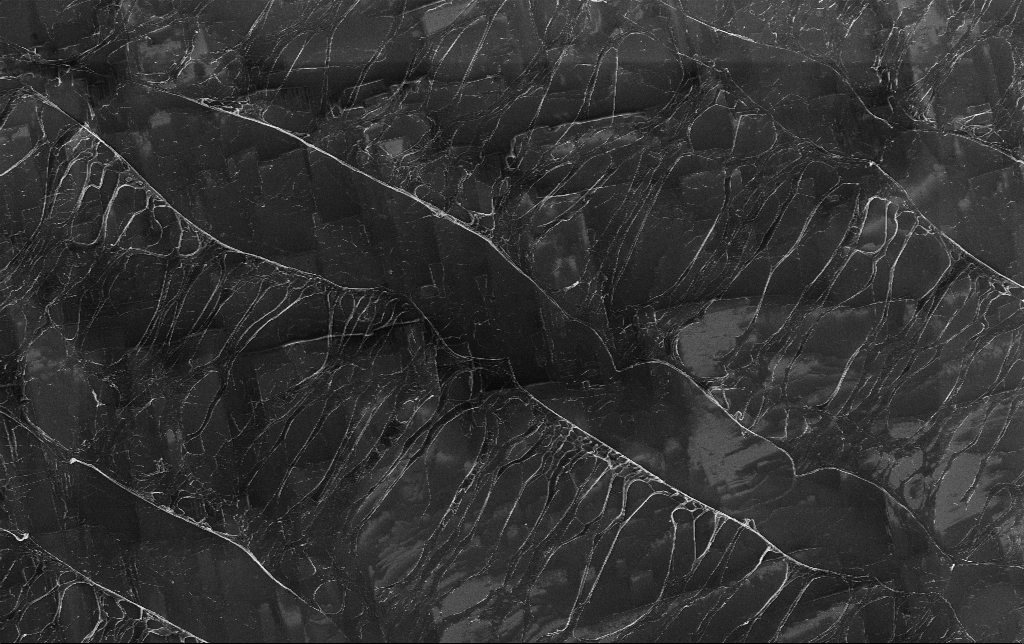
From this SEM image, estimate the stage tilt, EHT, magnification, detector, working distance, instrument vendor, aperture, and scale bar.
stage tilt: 0°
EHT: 5 kV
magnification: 1.98 K X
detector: InLens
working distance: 3.8 mm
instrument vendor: Zeiss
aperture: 30 µm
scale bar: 10000 nm